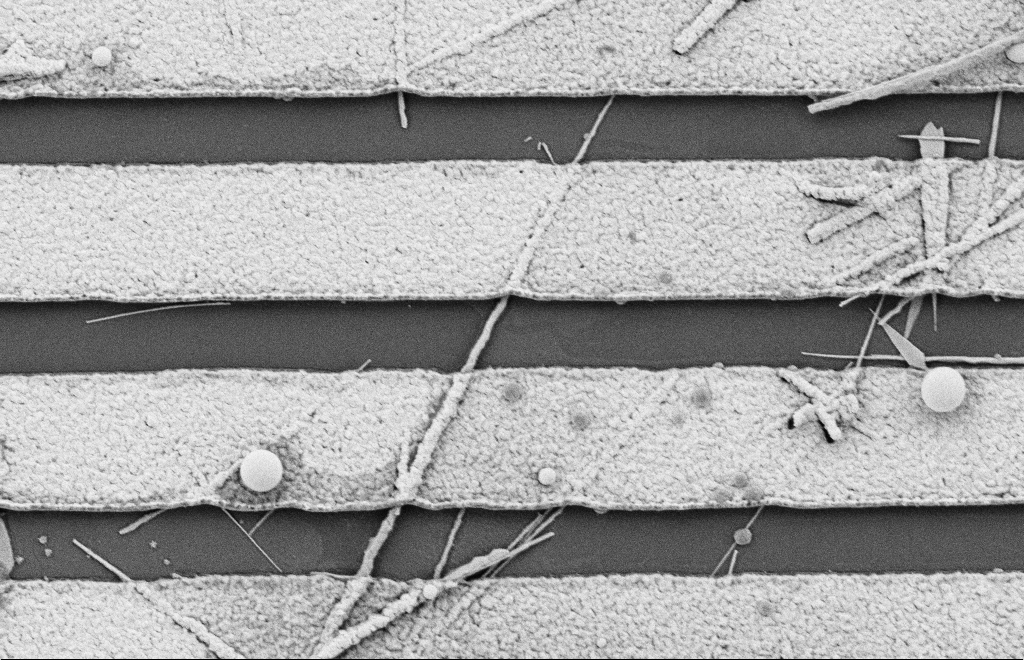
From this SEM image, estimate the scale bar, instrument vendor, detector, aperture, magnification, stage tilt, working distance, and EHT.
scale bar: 2000 nm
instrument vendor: Zeiss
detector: SE2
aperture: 20 µm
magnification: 18.92 K X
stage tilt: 0°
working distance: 10 mm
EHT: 2 kV